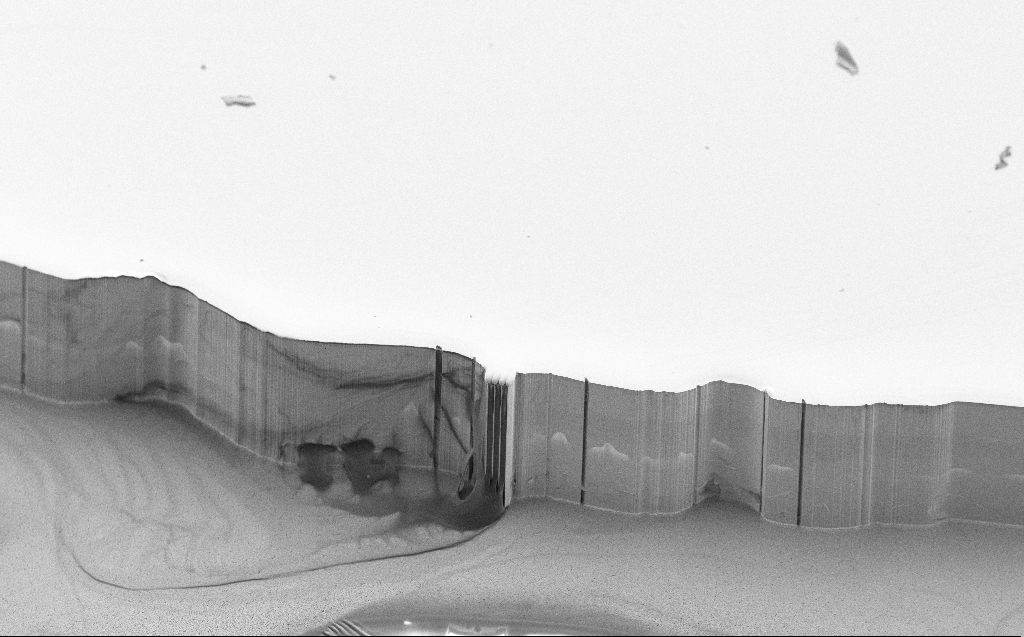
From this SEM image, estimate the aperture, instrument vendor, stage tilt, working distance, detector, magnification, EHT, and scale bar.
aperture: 30 µm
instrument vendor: Zeiss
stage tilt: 45°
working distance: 6 mm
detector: SE2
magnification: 0.222 K X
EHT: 10 kV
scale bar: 100000 nm